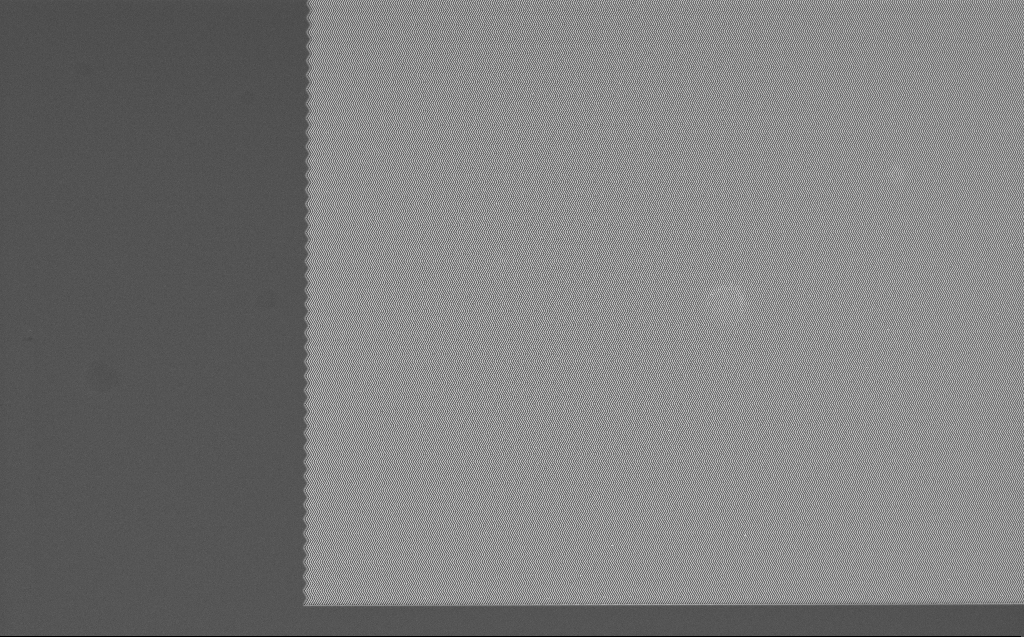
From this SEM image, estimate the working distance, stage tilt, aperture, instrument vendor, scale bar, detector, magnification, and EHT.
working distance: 7 mm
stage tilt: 0°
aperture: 30 µm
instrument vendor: Zeiss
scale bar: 10000 nm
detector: InLens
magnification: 2.65 K X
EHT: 5 kV